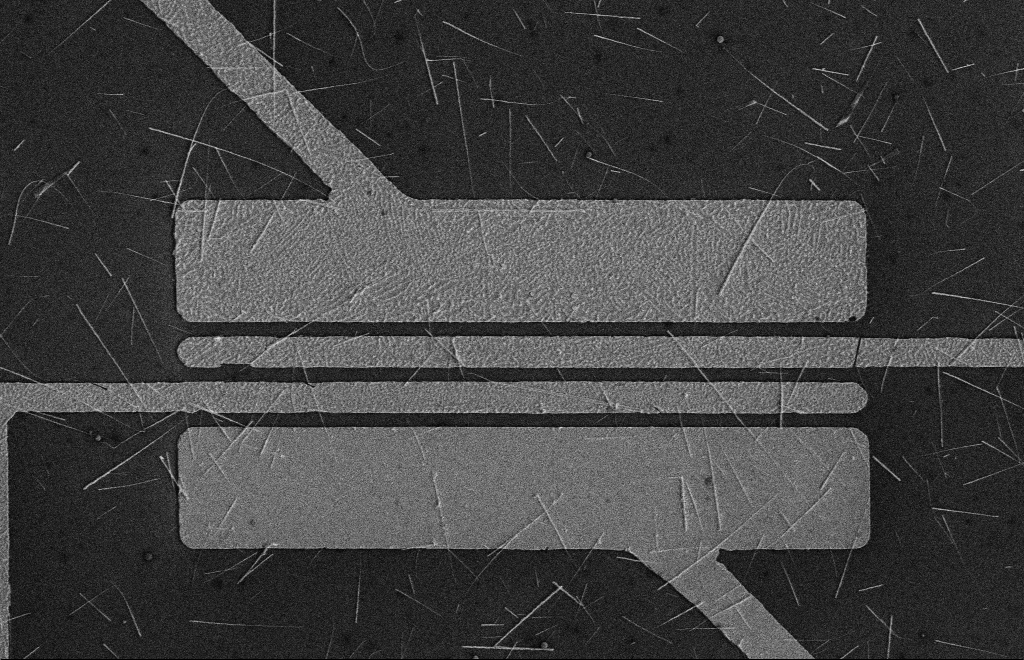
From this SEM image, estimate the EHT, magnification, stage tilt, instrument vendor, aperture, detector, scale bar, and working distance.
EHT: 5 kV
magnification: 4.2 K X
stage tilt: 0°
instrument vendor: Zeiss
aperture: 10 µm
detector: SE2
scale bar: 10000 nm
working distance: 16 mm